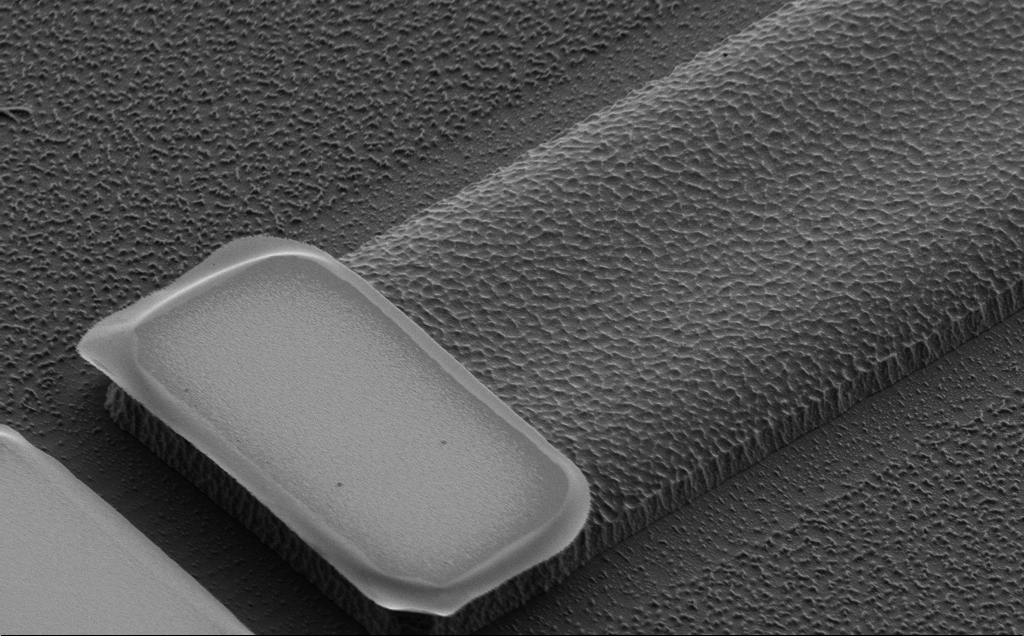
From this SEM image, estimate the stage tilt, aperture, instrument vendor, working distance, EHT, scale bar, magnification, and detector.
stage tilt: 43°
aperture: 30 µm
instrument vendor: Zeiss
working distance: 10 mm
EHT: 2 kV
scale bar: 2000 nm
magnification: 11.02 K X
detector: SE2